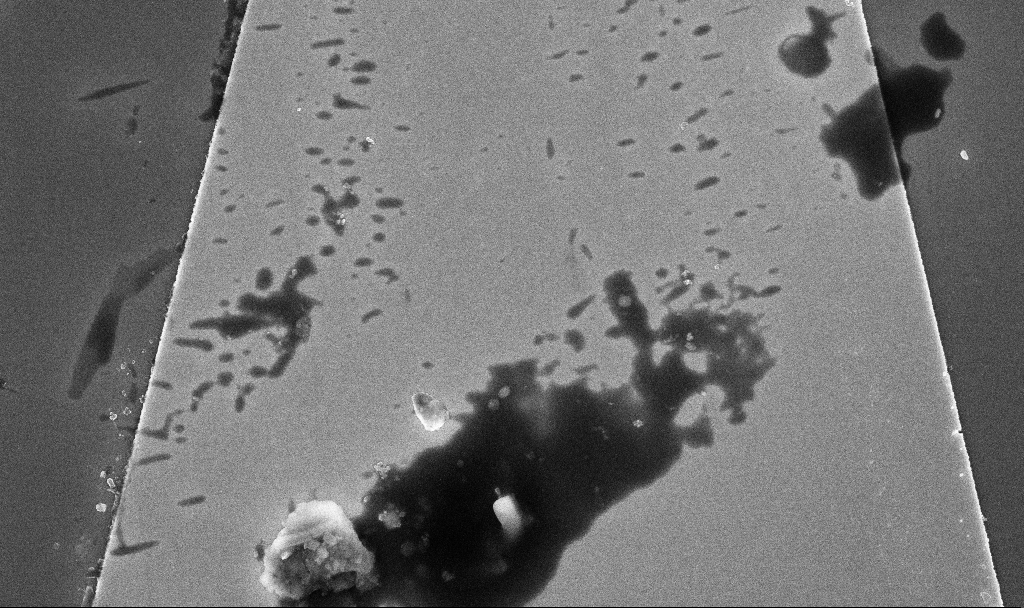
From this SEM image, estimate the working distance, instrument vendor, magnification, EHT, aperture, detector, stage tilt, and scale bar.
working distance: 10.3 mm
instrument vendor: Zeiss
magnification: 26.45 K X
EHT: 5 kV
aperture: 30 µm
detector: InLens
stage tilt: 0°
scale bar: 2000 nm